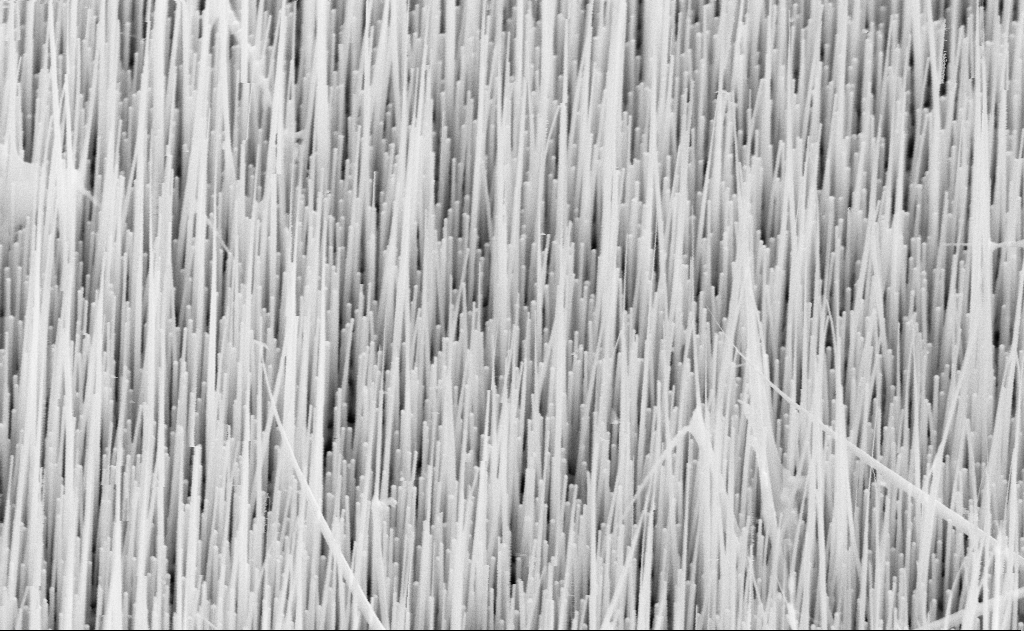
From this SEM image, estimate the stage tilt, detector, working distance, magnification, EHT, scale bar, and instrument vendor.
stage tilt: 45°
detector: SE2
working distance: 16 mm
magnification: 40 K X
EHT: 10 kV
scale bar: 1000 nm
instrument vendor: Zeiss